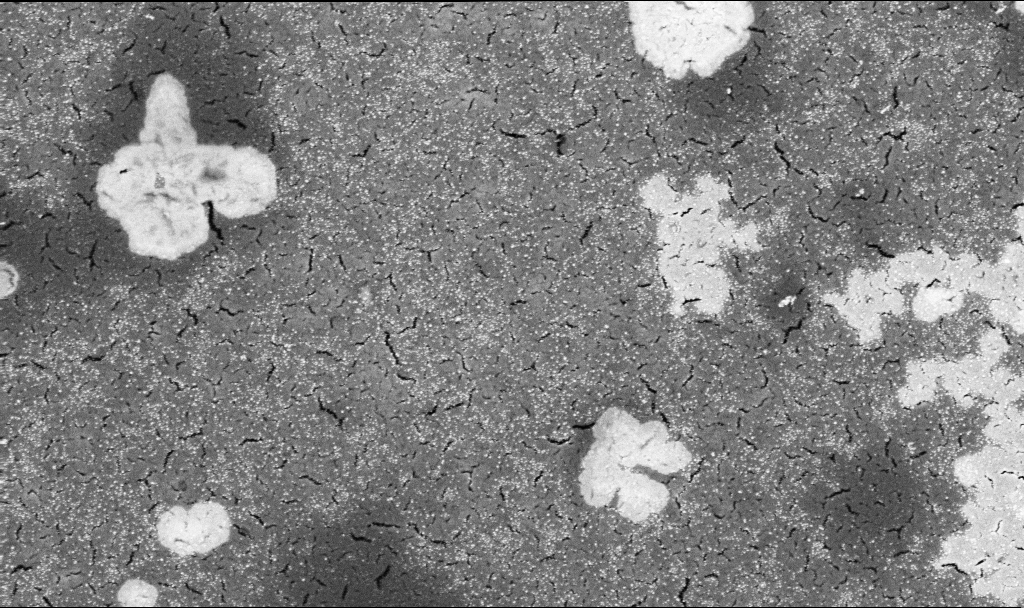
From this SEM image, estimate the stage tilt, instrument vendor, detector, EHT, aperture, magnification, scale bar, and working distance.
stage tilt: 0°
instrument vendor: Zeiss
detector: InLens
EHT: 5 kV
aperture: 30 µm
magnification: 31.04 K X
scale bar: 1000 nm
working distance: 3.3 mm